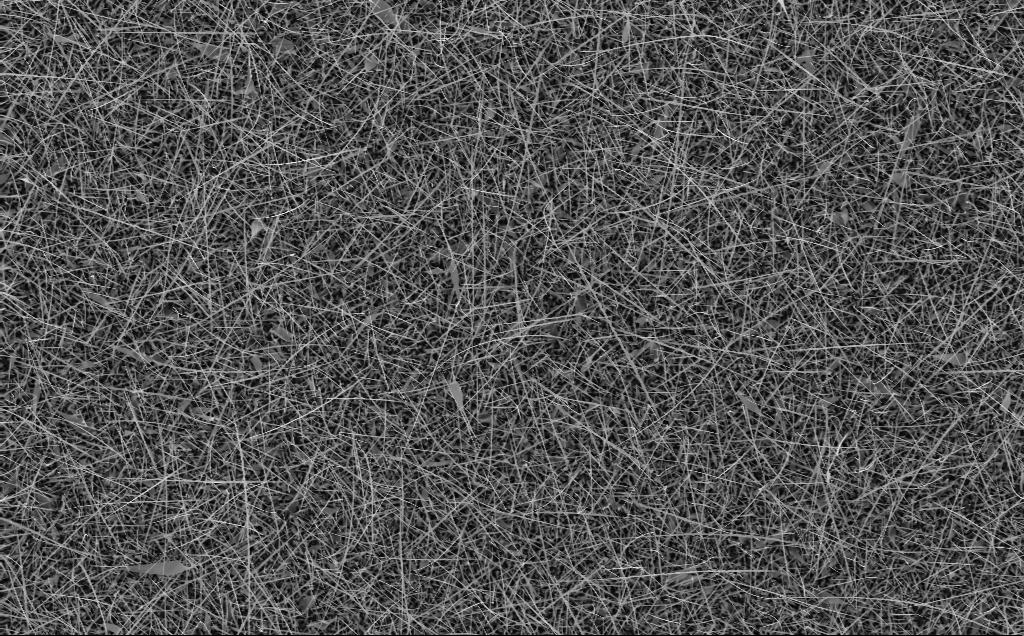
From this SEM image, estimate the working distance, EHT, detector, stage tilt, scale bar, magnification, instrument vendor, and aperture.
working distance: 5 mm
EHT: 10 kV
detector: InLens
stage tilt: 0°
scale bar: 10000 nm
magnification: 5 K X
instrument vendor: Zeiss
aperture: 30 µm